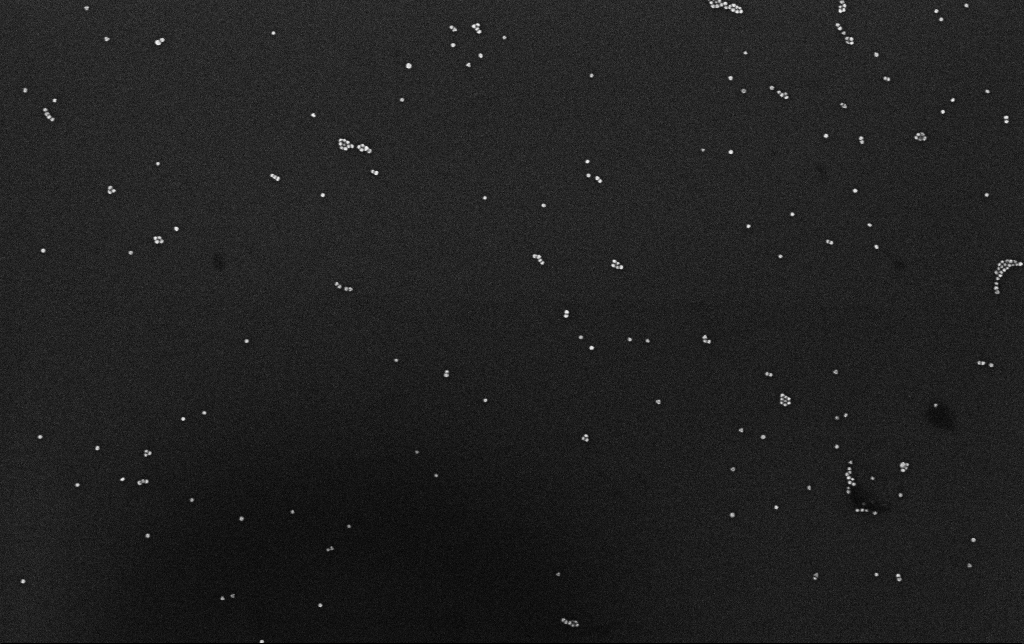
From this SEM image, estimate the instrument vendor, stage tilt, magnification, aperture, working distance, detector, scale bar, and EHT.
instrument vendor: Zeiss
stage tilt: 0°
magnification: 100 K X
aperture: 30 µm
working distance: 3.1 mm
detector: InLens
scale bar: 200 nm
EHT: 10 kV